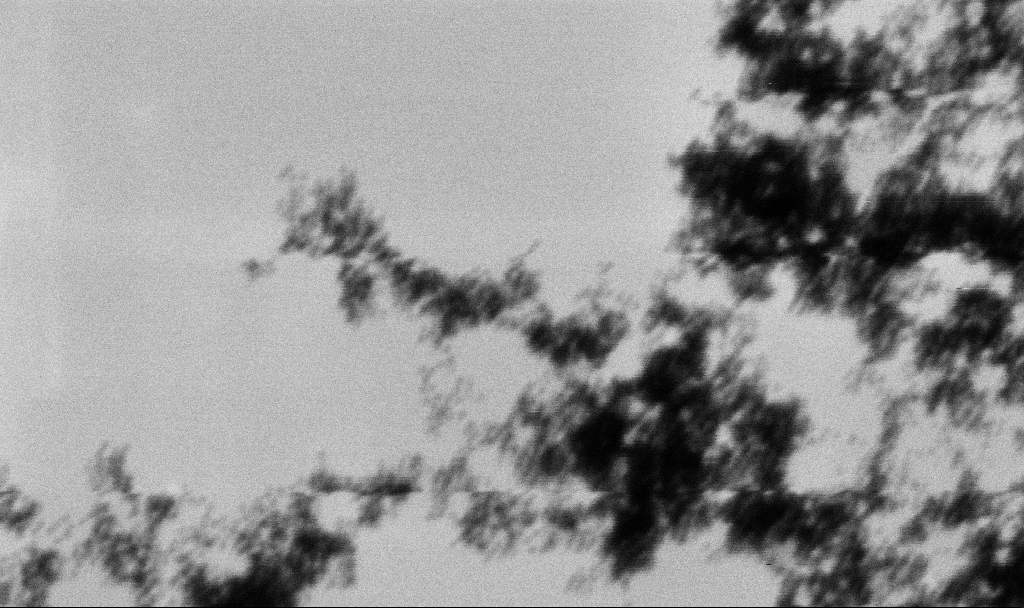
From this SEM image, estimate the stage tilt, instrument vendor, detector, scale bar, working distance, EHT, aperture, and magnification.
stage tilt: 0°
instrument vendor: Zeiss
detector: SE2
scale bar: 200 nm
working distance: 11.4 mm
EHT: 4 kV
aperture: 30 µm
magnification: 200 K X